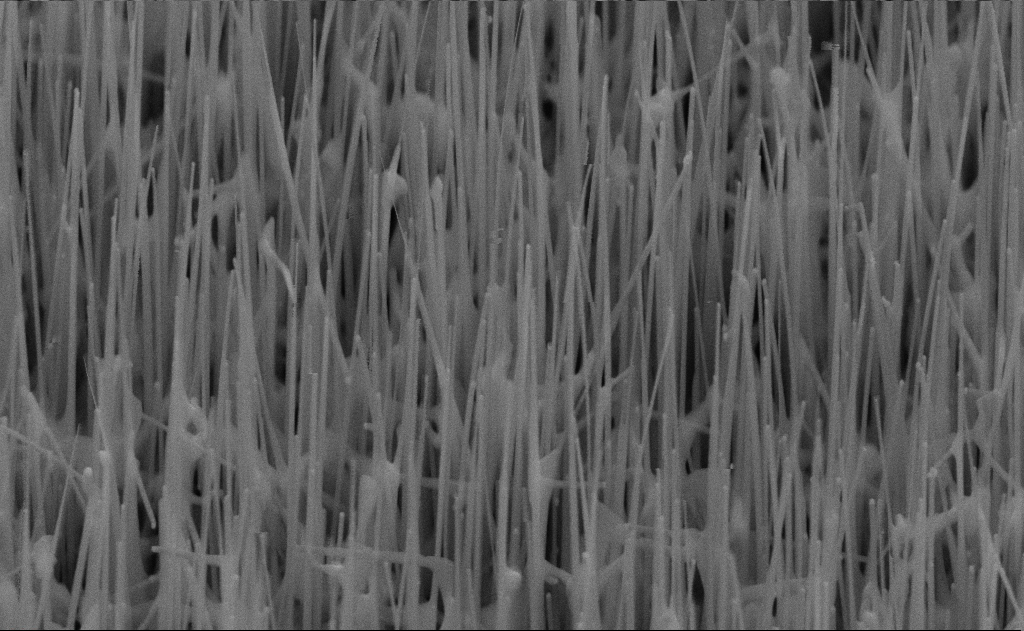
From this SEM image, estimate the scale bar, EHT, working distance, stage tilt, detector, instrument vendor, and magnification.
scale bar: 200 nm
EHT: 10 kV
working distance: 6 mm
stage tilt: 45°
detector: SE2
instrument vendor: Zeiss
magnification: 80 K X